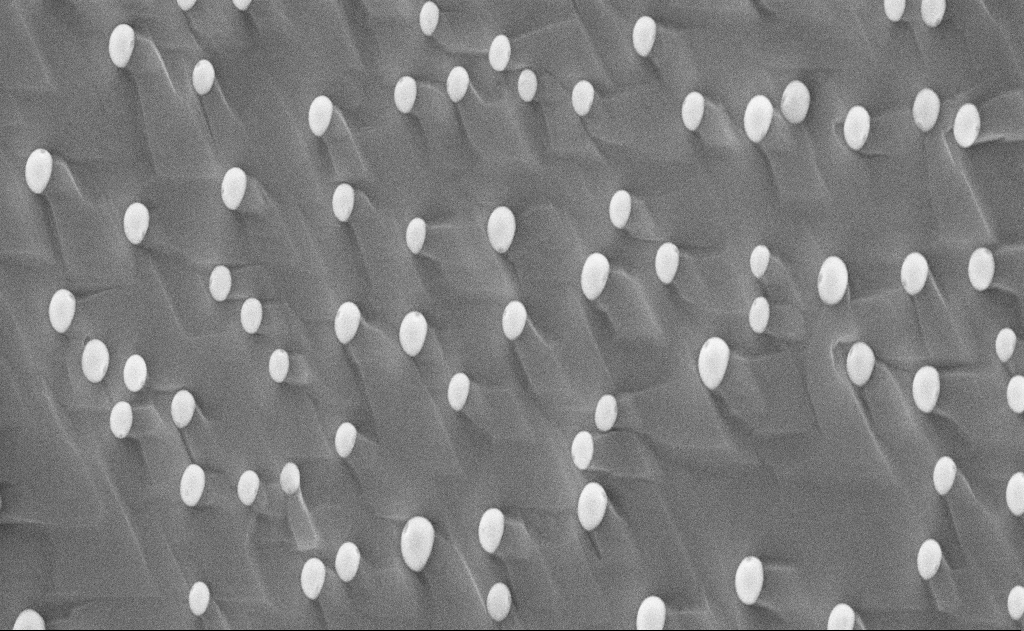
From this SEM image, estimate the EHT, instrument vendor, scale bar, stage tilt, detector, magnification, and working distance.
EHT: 10 kV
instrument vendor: Zeiss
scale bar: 1000 nm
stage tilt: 0°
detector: SE2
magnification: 19.72 K X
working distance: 12 mm